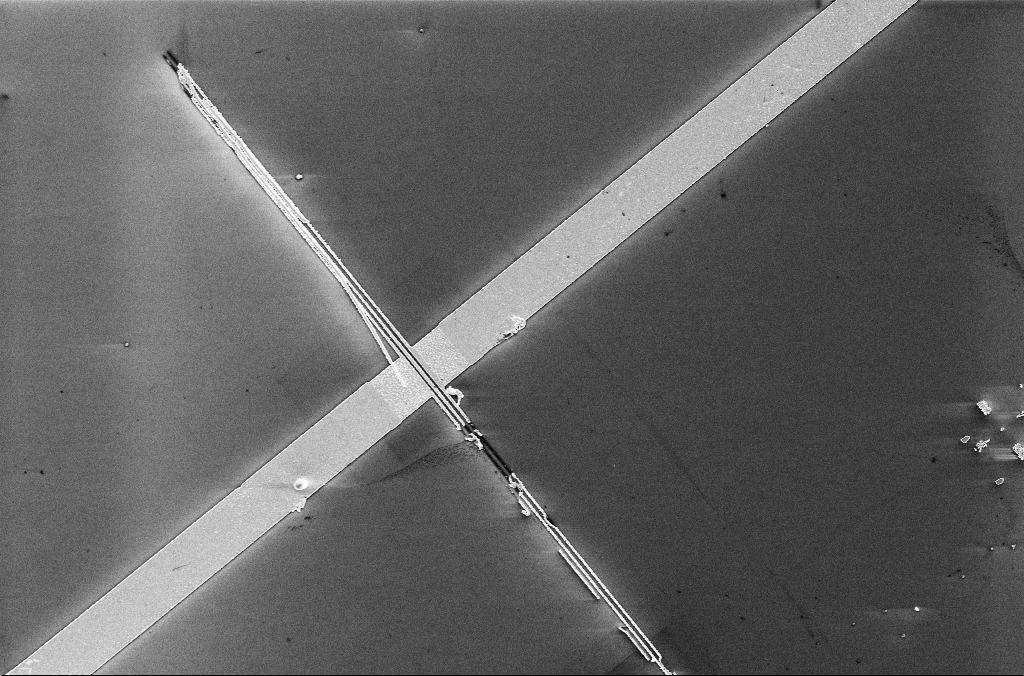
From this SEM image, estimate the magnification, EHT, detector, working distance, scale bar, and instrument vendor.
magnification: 3.83 K X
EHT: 5 kV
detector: InLens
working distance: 3.4 mm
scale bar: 10000 nm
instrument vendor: Zeiss